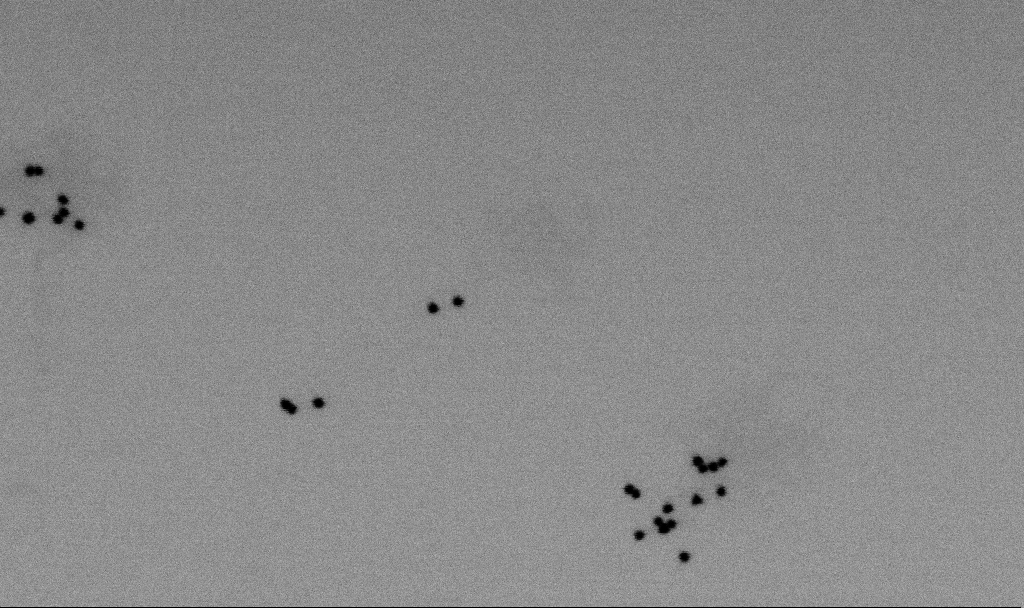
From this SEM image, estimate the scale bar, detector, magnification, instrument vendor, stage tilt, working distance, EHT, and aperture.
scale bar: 200 nm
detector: SE2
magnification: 153.48 K X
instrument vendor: Zeiss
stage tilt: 0°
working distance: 6.5 mm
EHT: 2 kV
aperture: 30 µm